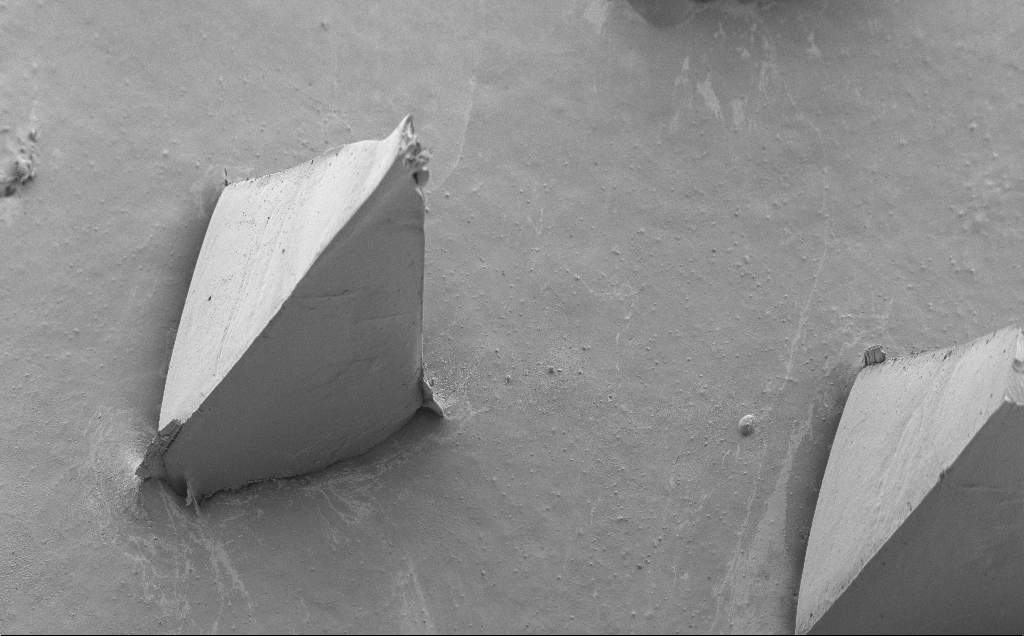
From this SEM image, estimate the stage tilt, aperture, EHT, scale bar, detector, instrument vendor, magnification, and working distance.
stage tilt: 40°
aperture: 30 µm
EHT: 5 kV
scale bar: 100000 nm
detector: SE2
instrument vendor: Zeiss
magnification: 0.158 K X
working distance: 8 mm